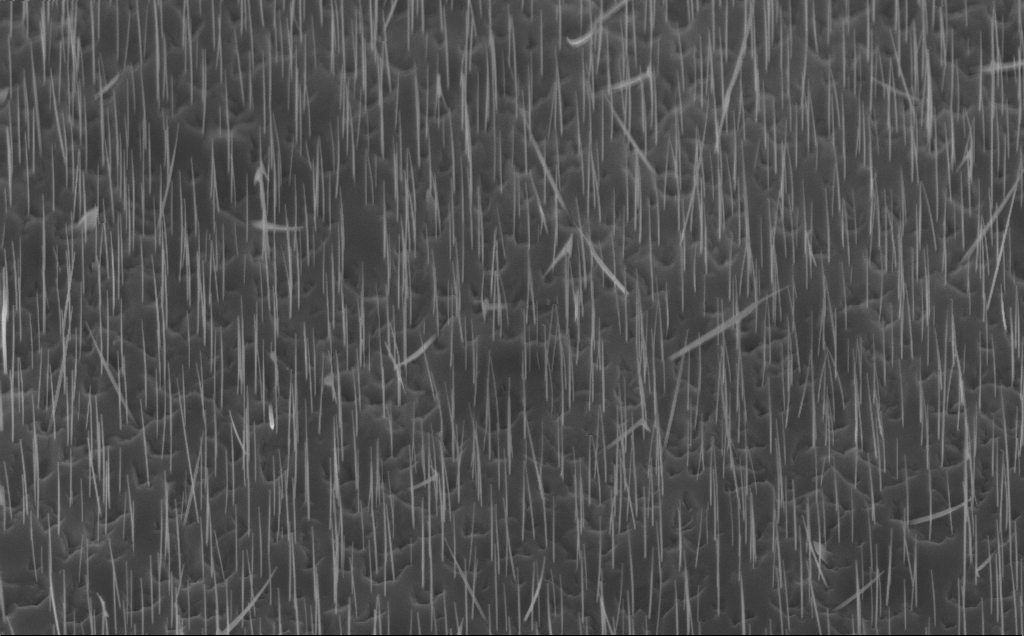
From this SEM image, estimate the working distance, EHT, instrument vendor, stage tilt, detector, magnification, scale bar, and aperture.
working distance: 7 mm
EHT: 10 kV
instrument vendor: Zeiss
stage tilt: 45°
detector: InLens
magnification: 20 K X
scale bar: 1000 nm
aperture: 30 µm